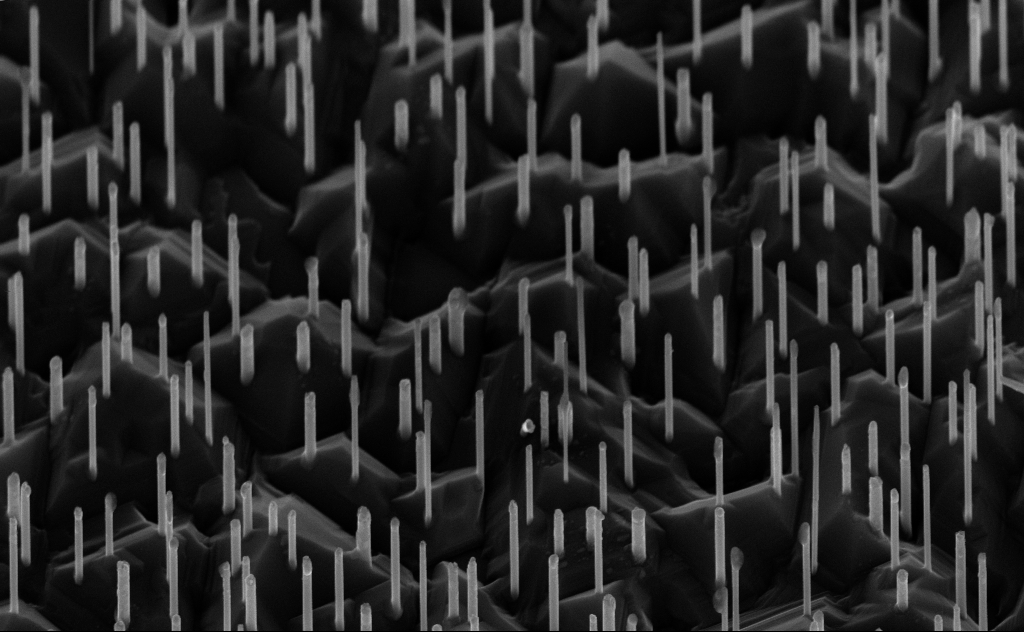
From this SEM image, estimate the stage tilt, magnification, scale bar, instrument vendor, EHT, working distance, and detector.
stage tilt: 45°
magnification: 40 K X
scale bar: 1000 nm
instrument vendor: Zeiss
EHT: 10 kV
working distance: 6 mm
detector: InLens